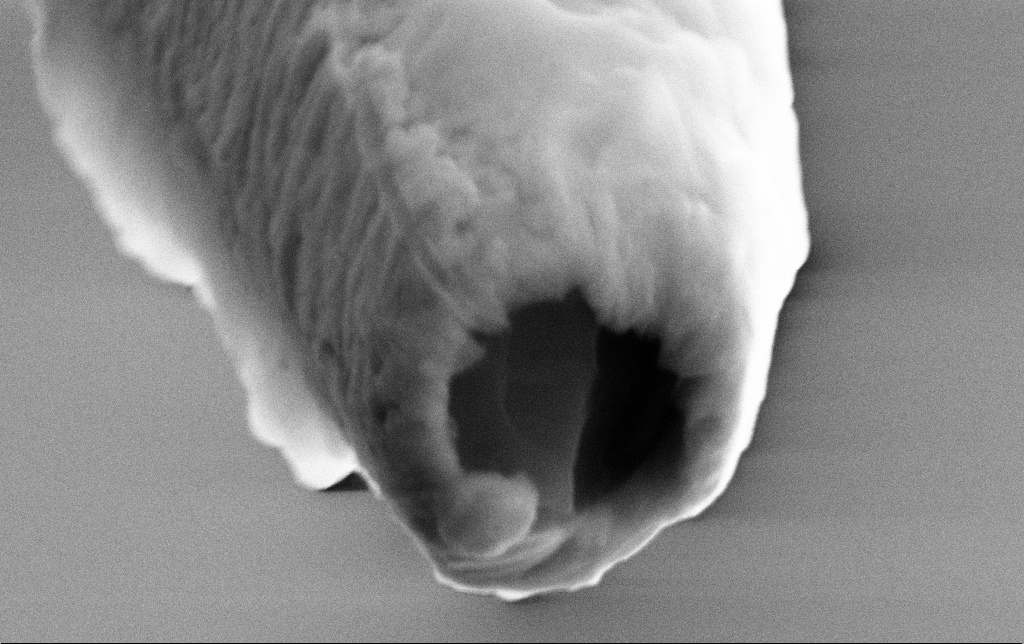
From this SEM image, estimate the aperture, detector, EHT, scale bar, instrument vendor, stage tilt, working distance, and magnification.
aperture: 30 µm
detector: SE2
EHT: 10 kV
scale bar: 200 nm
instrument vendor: Zeiss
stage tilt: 70°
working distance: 6.2 mm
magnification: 116.91 K X